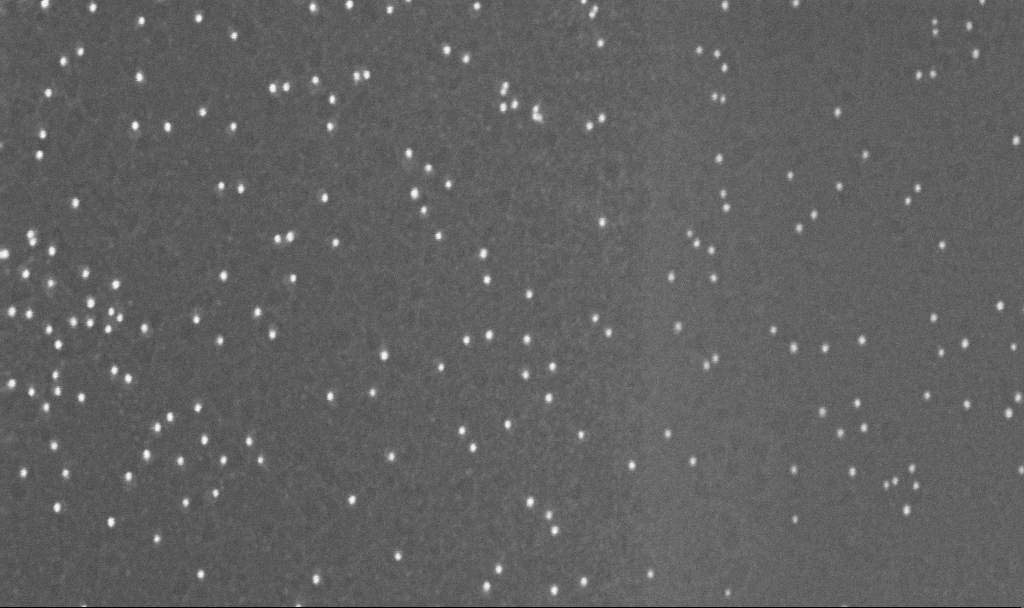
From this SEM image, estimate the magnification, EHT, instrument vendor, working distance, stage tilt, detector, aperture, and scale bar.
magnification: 200 K X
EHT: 10 kV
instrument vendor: Zeiss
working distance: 3.2 mm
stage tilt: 0°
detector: InLens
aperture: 30 µm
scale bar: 100 nm